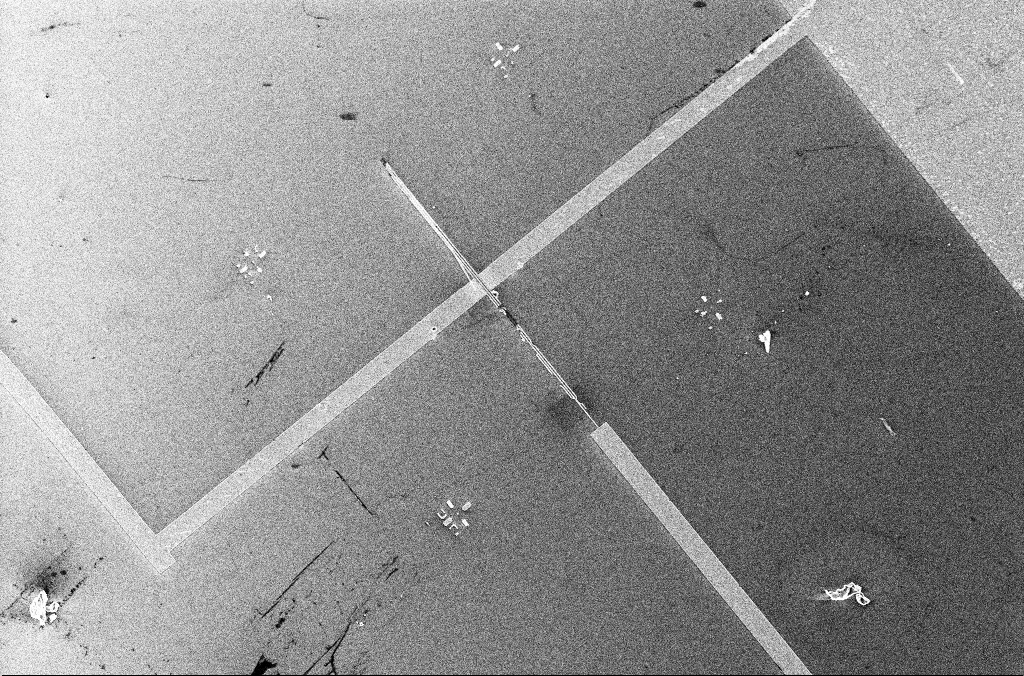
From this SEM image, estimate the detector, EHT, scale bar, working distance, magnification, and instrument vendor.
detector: InLens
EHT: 5 kV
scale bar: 30000 nm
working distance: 3.3 mm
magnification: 1.51 K X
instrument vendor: Zeiss